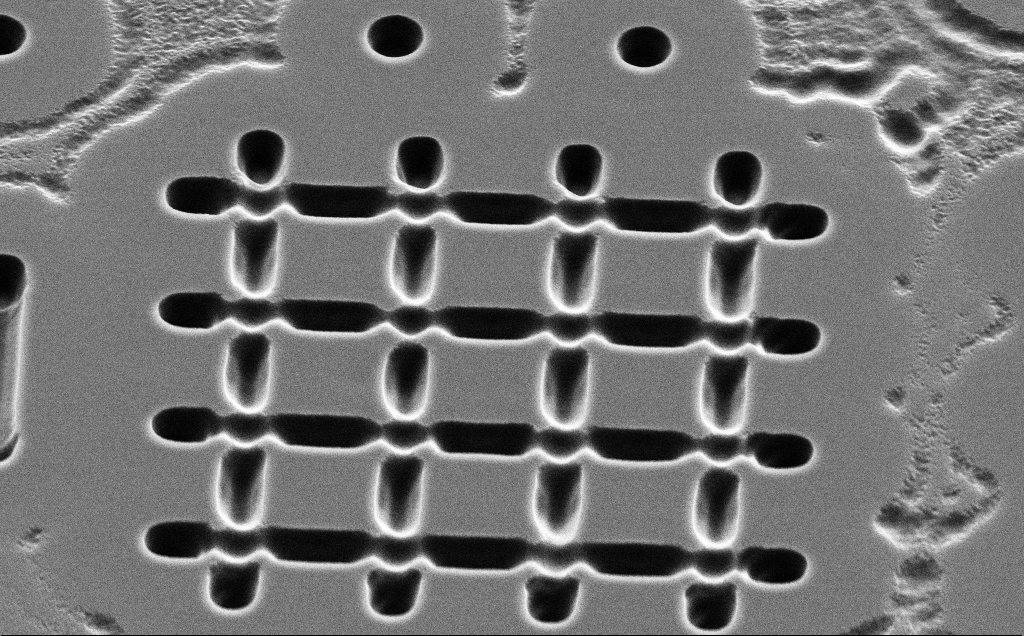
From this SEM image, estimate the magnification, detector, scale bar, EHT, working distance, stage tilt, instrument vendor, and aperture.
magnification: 9.09 K X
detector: SE2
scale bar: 2000 nm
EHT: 5 kV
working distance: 11 mm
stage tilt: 45°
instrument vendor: Zeiss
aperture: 30 µm